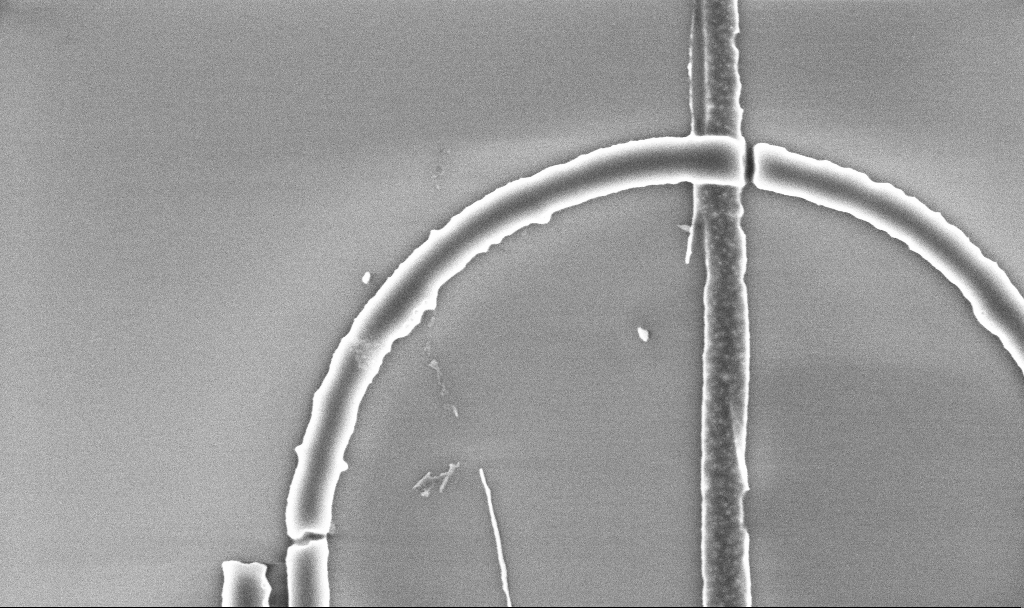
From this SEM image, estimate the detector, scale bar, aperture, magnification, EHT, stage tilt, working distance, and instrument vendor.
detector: InLens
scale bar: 1000 nm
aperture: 30 µm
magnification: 30.68 K X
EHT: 5 kV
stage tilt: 0°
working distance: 5.2 mm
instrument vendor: Zeiss